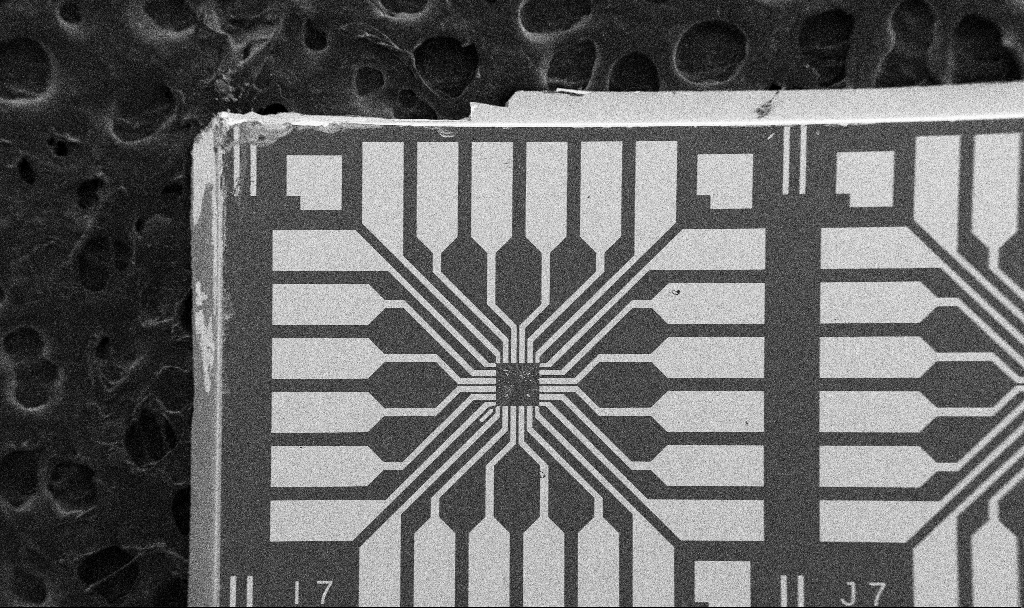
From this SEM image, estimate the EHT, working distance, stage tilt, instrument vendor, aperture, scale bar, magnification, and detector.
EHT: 5 kV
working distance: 10.7 mm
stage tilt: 0°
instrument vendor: Zeiss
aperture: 30 µm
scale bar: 200000 nm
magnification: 0.1 K X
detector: SE2